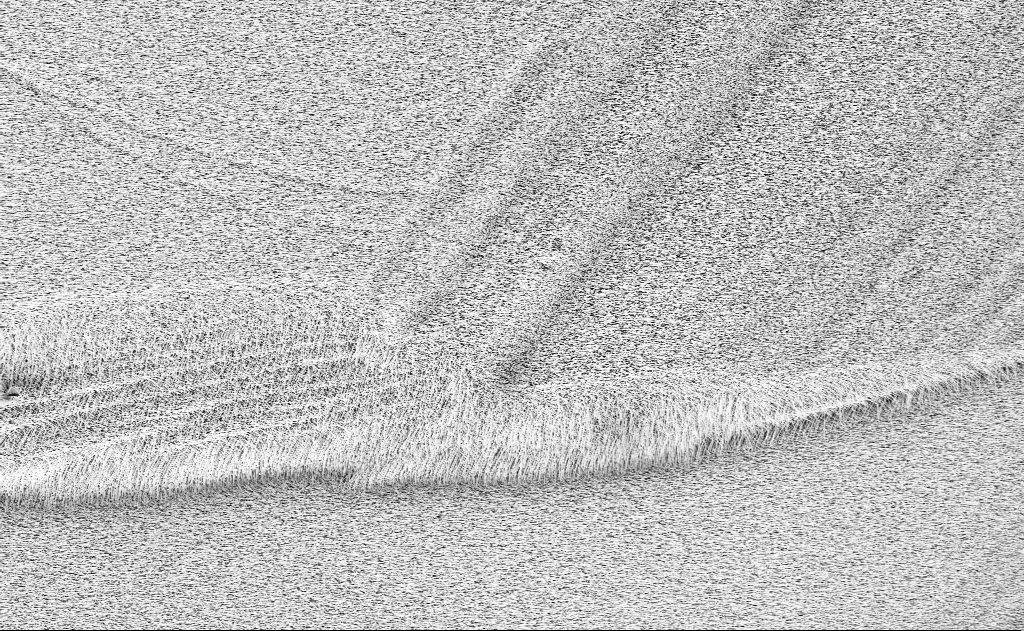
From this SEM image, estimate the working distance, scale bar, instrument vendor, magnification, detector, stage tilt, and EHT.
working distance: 13 mm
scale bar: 10000 nm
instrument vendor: Zeiss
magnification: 2 K X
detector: InLens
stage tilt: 0°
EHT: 10 kV